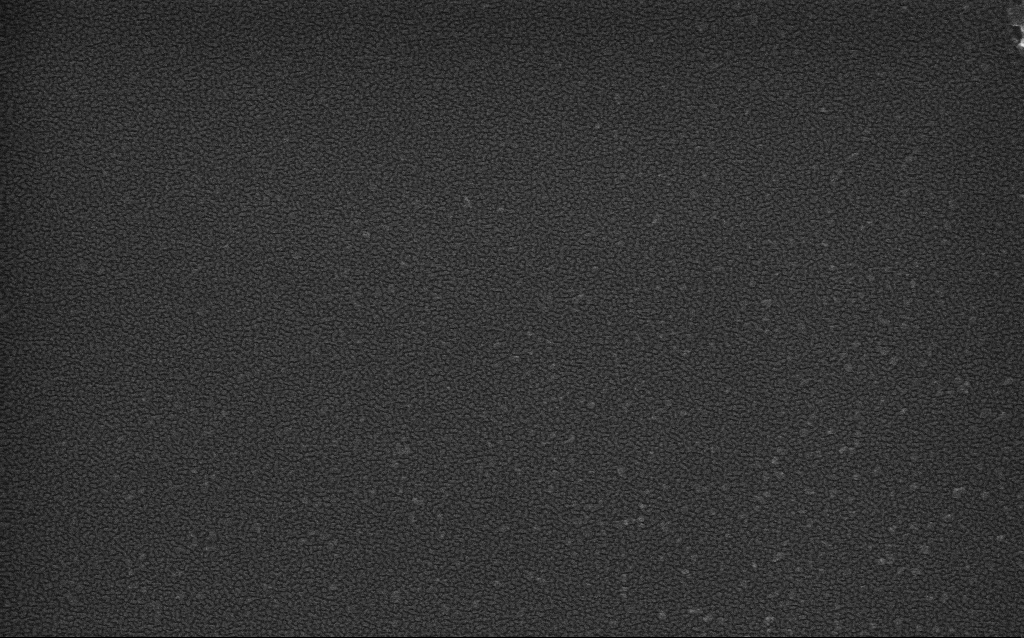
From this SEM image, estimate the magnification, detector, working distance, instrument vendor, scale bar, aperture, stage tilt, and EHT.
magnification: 100 K X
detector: InLens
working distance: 1.6 mm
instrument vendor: Zeiss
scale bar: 200 nm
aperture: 30 µm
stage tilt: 0°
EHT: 4 kV